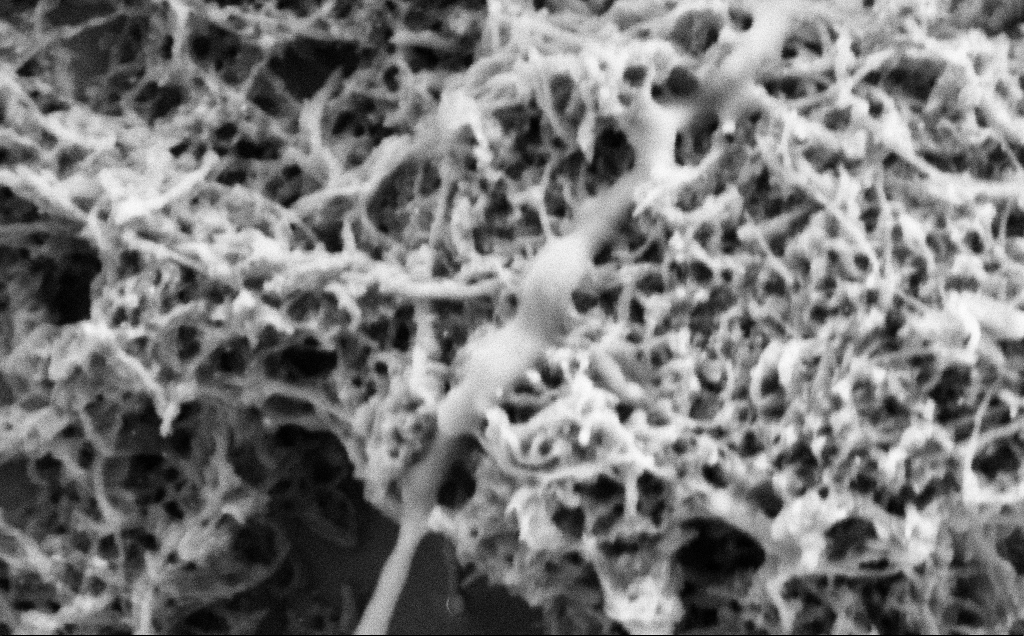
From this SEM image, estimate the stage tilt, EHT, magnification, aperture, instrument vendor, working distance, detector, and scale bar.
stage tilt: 0°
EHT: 2 kV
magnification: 100 K X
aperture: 30 µm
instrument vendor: Zeiss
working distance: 7.1 mm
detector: SE2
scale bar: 200 nm